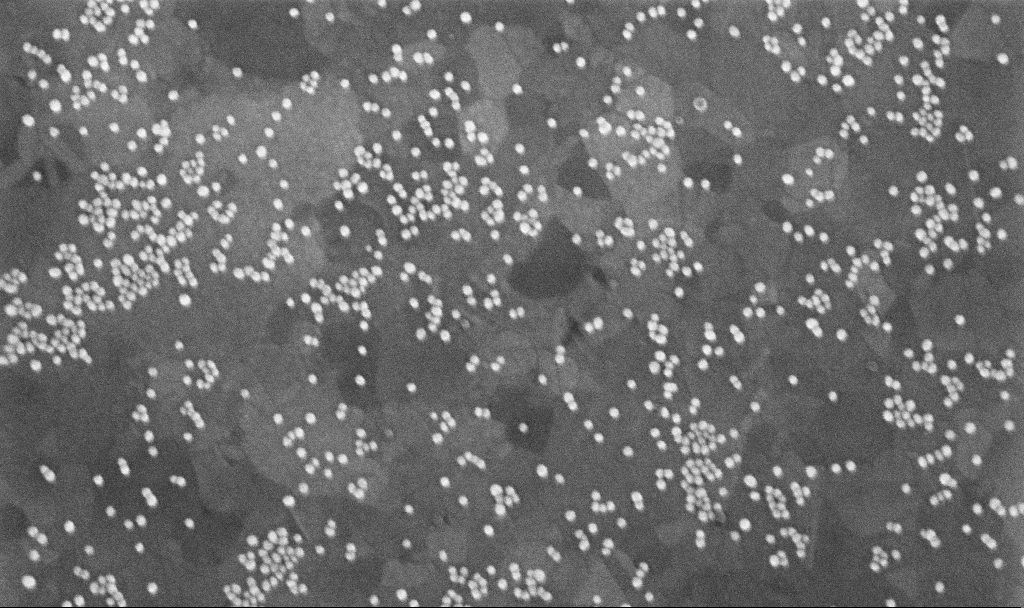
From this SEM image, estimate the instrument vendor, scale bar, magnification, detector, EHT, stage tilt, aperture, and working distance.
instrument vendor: Zeiss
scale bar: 200 nm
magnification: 177.15 K X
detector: InLens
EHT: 10 kV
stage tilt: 0°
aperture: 30 µm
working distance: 3.7 mm